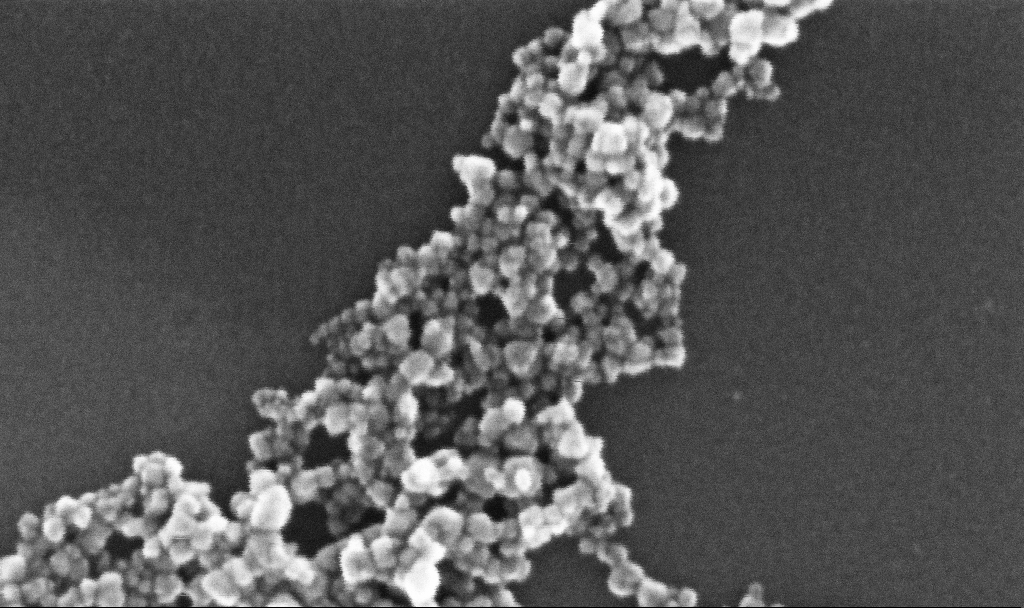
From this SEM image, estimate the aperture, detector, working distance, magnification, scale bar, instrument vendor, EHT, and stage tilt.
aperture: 30 µm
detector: InLens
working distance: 5.2 mm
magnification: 481.27 K X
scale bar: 100 nm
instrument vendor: Zeiss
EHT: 10 kV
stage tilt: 0°